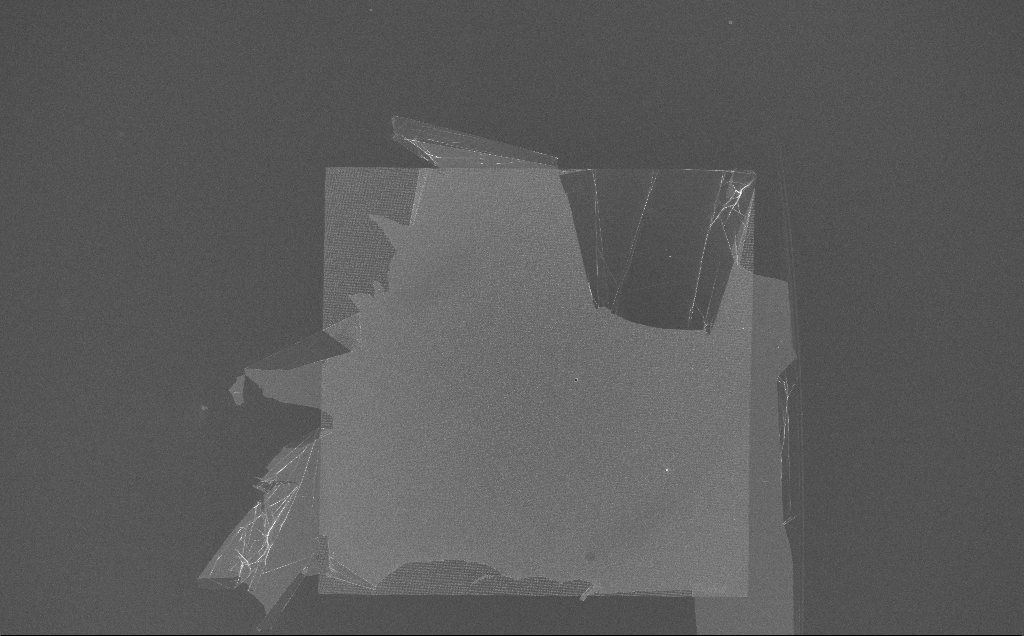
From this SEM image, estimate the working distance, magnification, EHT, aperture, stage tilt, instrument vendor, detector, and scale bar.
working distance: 7 mm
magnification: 0.212 K X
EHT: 10 kV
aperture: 30 µm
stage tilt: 0°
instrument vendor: Zeiss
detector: InLens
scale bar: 100000 nm